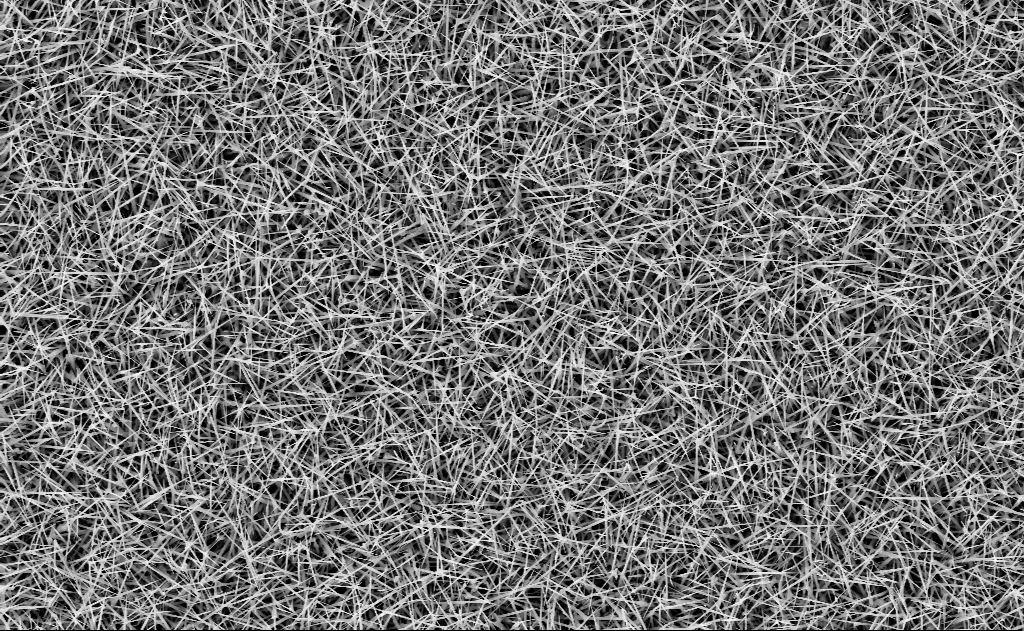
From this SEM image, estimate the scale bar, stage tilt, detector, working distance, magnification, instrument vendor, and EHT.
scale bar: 2000 nm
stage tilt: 0°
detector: InLens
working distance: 15 mm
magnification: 10 K X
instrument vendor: Zeiss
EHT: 10 kV